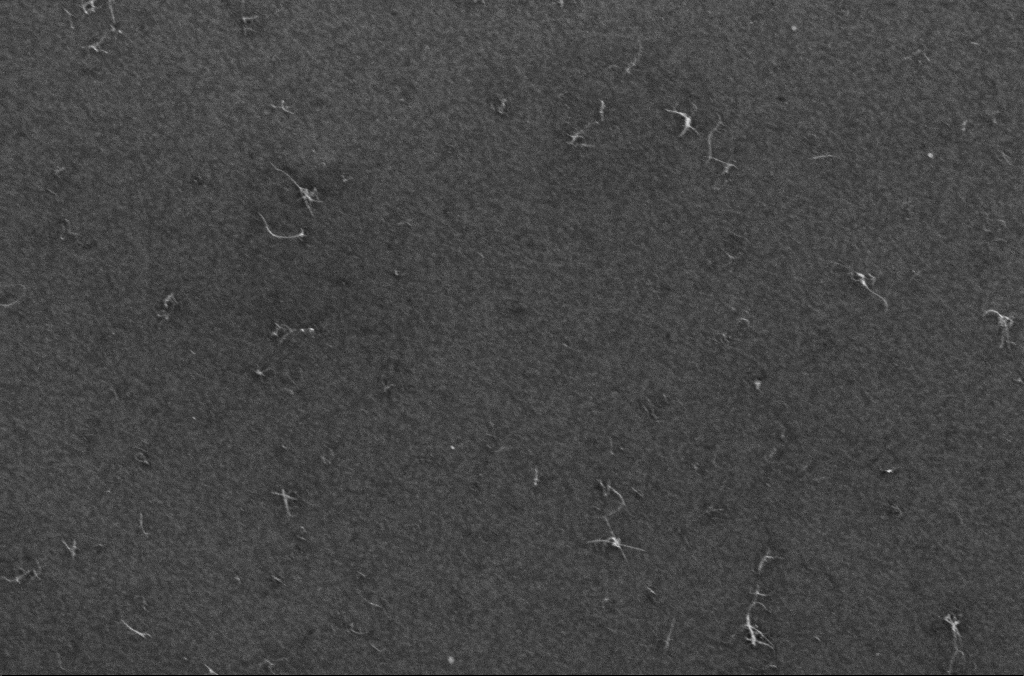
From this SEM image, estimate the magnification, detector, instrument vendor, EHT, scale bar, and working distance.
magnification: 101.76 K X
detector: InLens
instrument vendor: Zeiss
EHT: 10 kV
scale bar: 200 nm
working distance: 3.2 mm